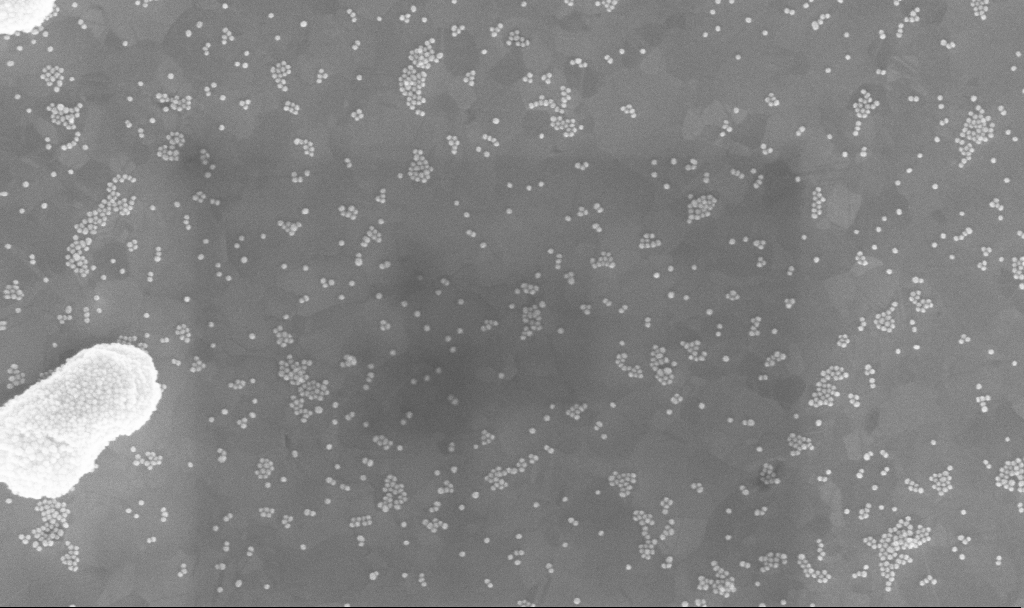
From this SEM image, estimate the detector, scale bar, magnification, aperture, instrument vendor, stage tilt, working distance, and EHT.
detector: InLens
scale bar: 200 nm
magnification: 115.58 K X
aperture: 30 µm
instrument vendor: Zeiss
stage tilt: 0°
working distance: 3.9 mm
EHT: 10 kV